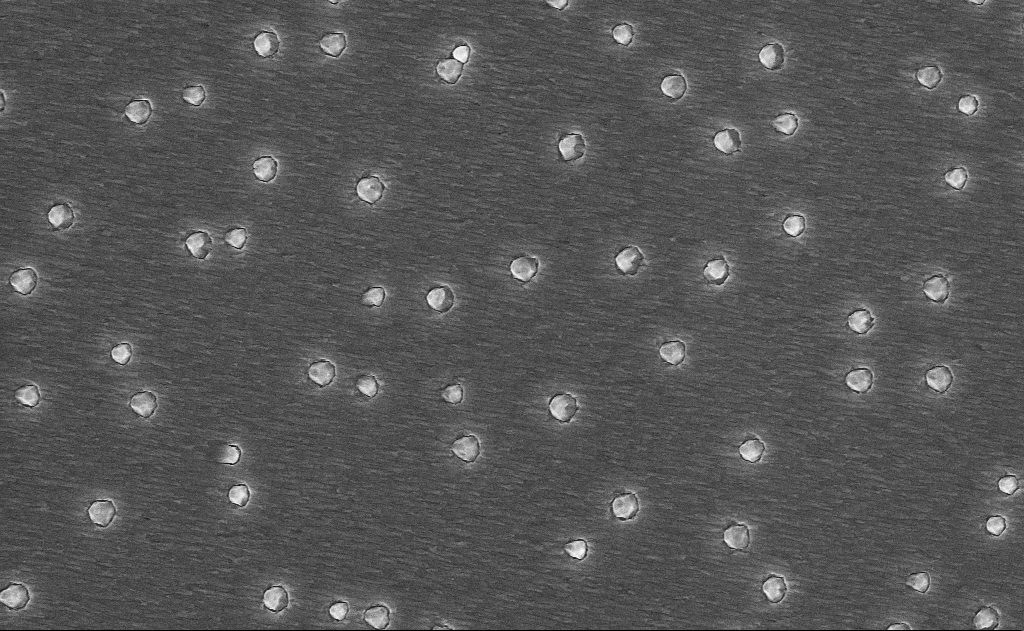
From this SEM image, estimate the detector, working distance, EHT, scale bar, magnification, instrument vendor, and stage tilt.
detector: InLens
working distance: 16 mm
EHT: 10 kV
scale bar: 2000 nm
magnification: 20 K X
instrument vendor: Zeiss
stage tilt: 0°